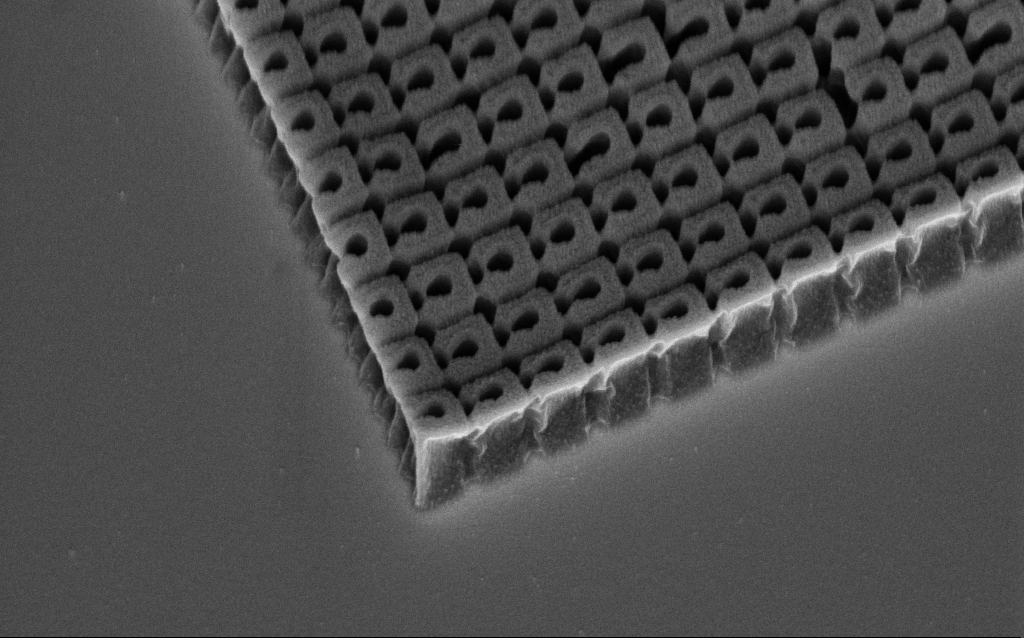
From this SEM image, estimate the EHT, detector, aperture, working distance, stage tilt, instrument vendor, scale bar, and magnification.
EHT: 3 kV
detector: InLens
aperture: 30 µm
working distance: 7.3 mm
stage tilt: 45°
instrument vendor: Zeiss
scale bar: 1000 nm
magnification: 54.2 K X